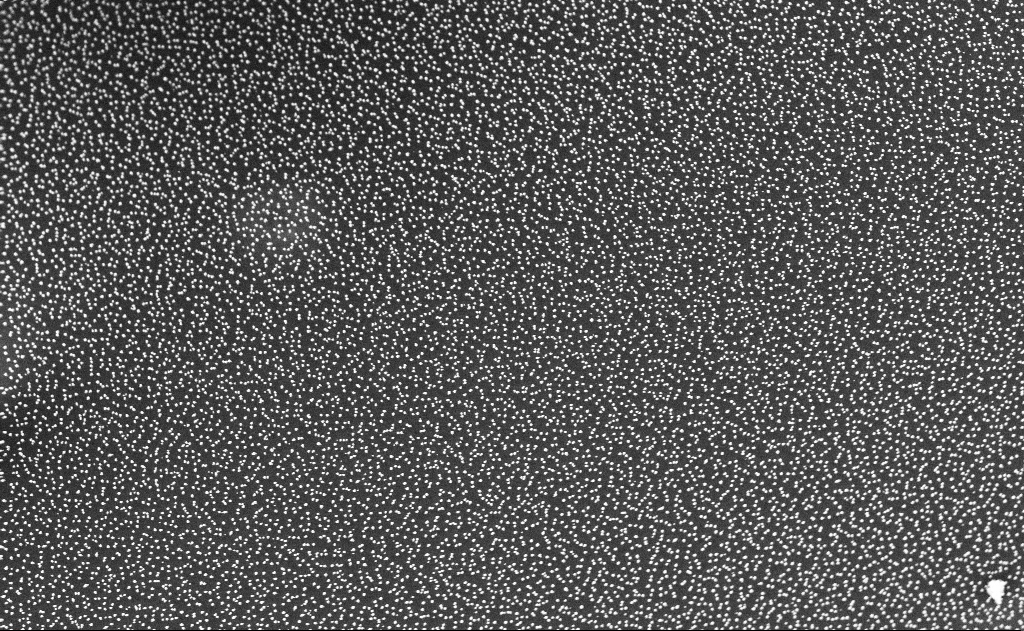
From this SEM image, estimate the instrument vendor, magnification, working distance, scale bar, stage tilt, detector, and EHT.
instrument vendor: Zeiss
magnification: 20 K X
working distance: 12 mm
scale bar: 2000 nm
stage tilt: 0°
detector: InLens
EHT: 10 kV